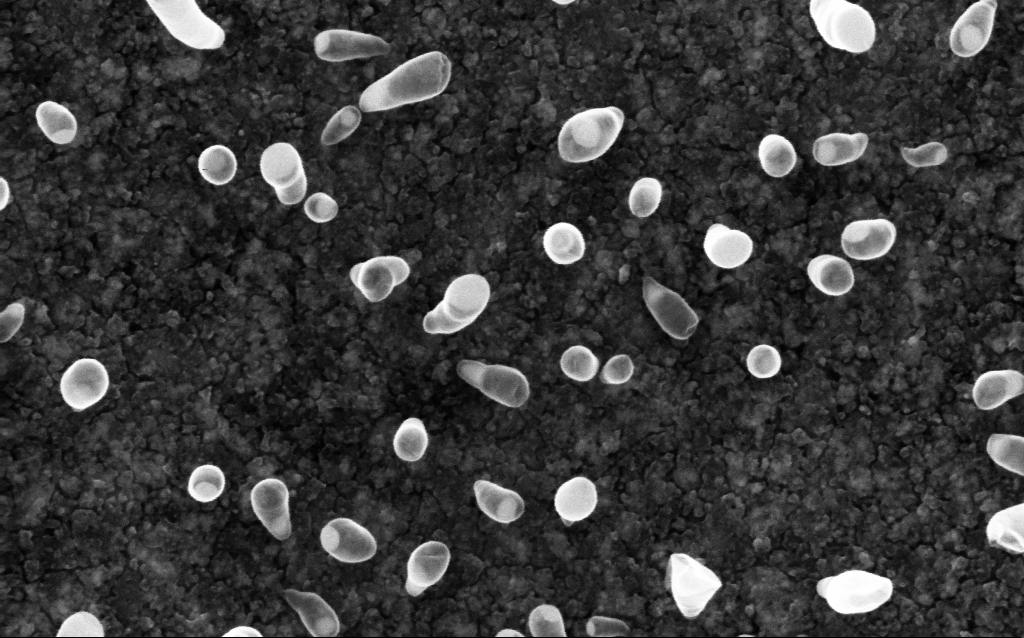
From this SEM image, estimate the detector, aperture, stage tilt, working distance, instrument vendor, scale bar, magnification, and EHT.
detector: InLens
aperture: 30 µm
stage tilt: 0°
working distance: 1.9 mm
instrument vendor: Zeiss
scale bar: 100 nm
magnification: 200 K X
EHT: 5 kV